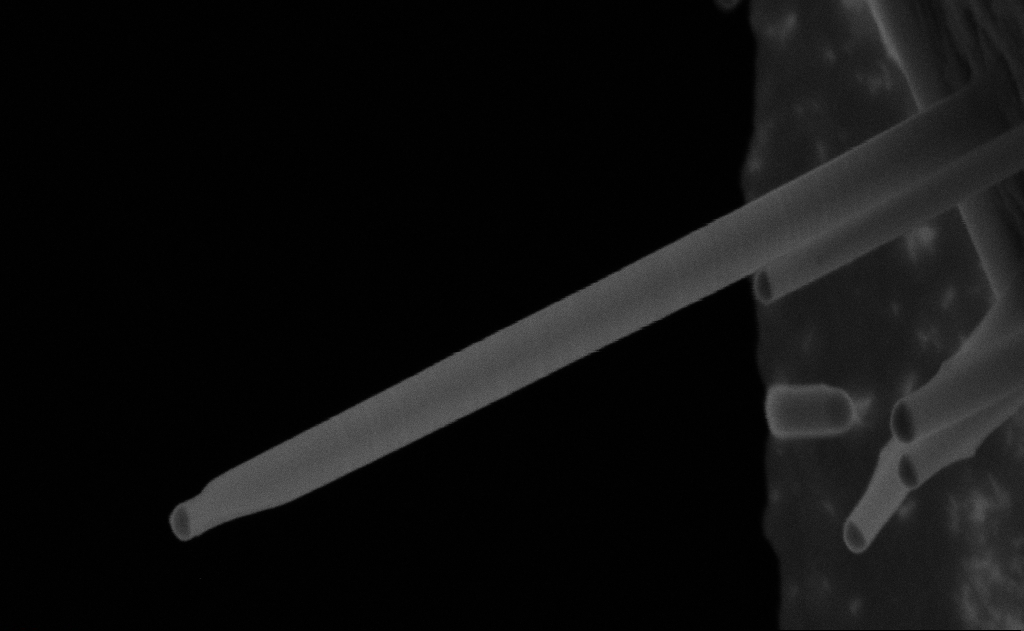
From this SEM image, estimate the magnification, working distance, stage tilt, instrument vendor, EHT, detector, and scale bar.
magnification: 203.08 K X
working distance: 9 mm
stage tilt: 0°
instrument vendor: Zeiss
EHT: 20 kV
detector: SE2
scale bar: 100 nm